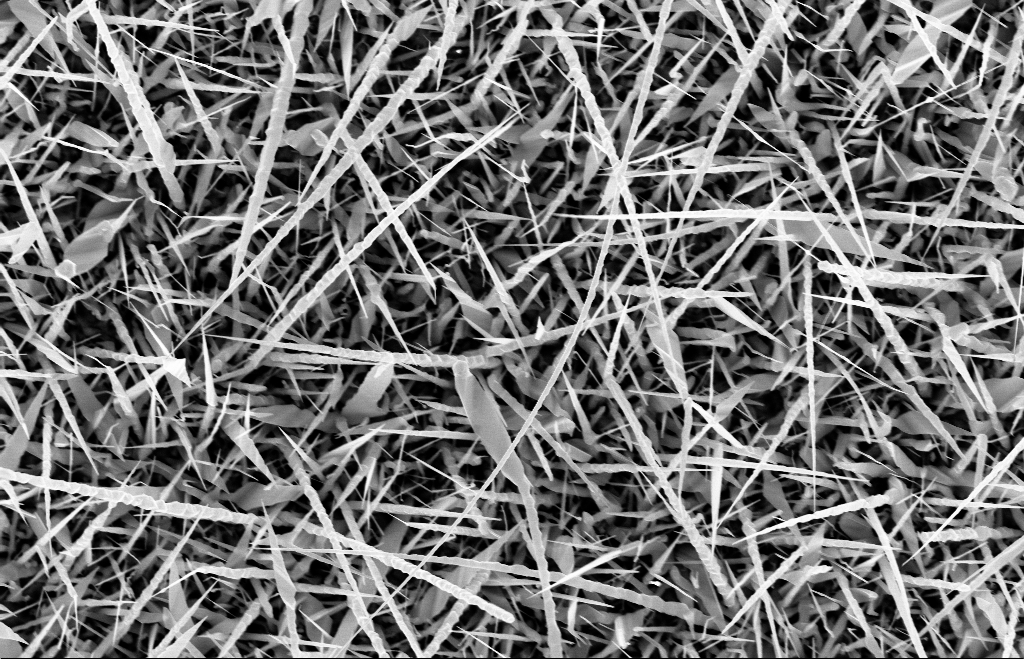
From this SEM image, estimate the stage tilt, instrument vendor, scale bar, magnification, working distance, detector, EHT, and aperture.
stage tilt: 0°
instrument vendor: Zeiss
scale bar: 1000 nm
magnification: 20 K X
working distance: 9 mm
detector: InLens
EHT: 10 kV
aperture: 30 µm